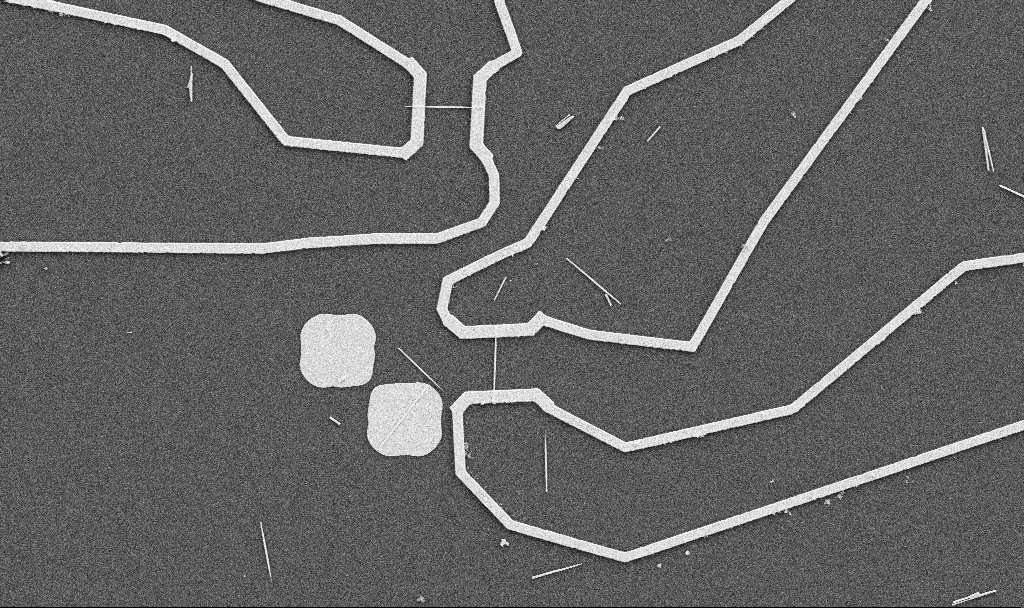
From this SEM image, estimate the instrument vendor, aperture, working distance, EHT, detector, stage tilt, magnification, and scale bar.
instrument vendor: Zeiss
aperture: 30 µm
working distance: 10.7 mm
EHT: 5 kV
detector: SE2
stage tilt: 0°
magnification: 5 K X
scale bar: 10000 nm